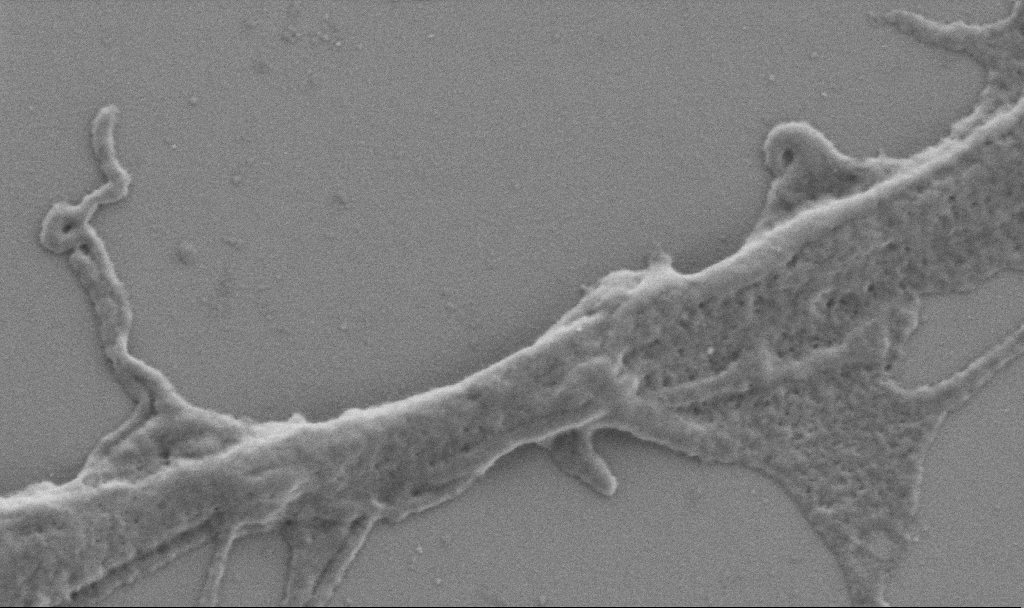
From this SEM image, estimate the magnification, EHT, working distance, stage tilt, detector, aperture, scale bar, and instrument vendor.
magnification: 50 K X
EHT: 0.9 kV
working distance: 6.9 mm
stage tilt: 0°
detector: SE2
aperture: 30 µm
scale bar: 1000 nm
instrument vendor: Zeiss